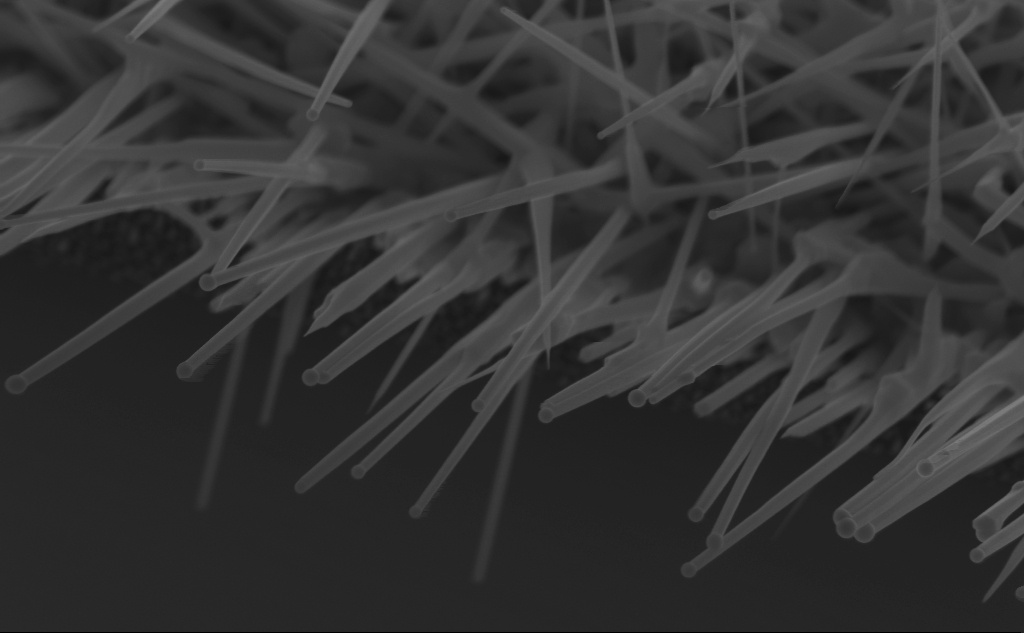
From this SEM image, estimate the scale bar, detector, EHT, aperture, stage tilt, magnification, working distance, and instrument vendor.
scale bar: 1000 nm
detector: InLens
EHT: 10 kV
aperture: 30 µm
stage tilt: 45°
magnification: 58.64 K X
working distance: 5 mm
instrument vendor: Zeiss